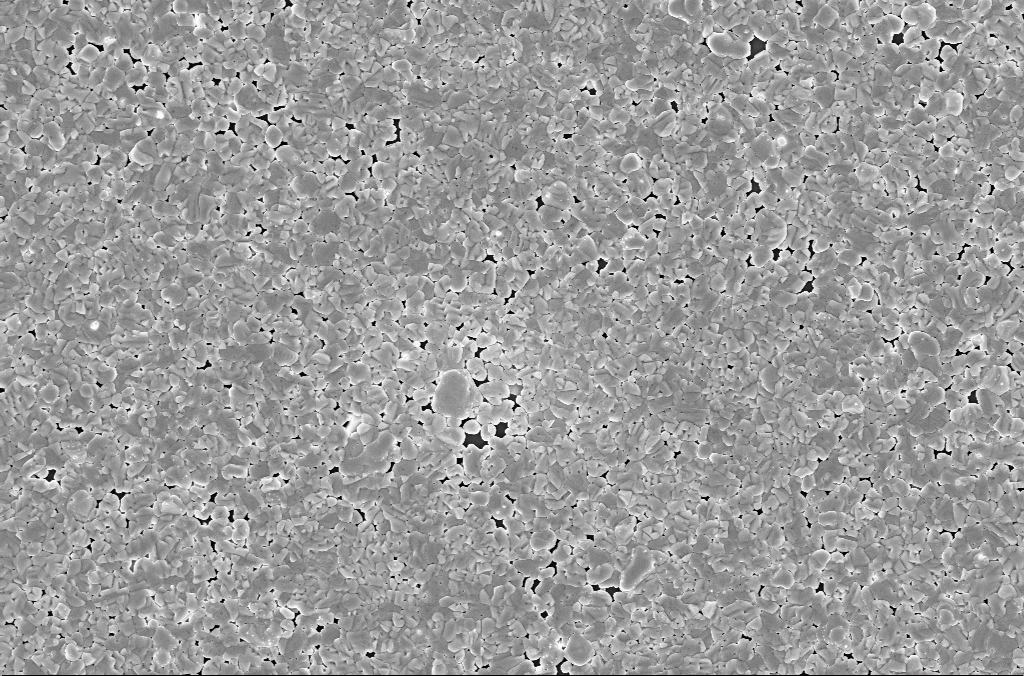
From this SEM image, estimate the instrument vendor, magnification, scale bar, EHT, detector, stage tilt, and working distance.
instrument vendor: Zeiss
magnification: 10 K X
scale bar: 2000 nm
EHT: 5 kV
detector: InLens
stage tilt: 0°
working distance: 3 mm